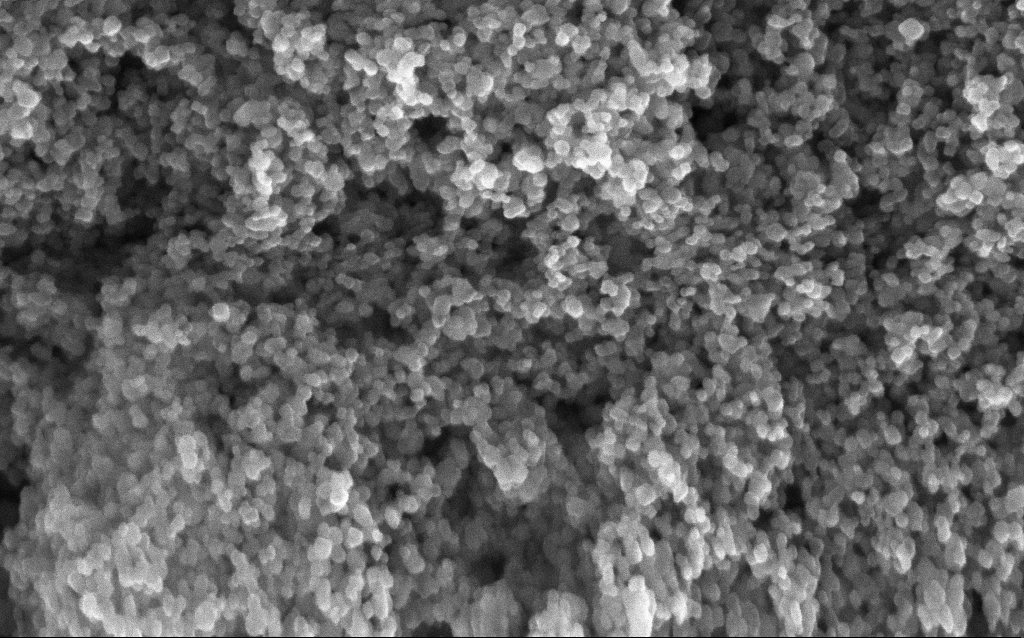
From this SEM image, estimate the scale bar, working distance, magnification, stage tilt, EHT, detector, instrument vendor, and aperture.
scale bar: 100 nm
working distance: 2.8 mm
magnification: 211.33 K X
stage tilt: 0°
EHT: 10 kV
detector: InLens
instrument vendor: Zeiss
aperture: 30 µm